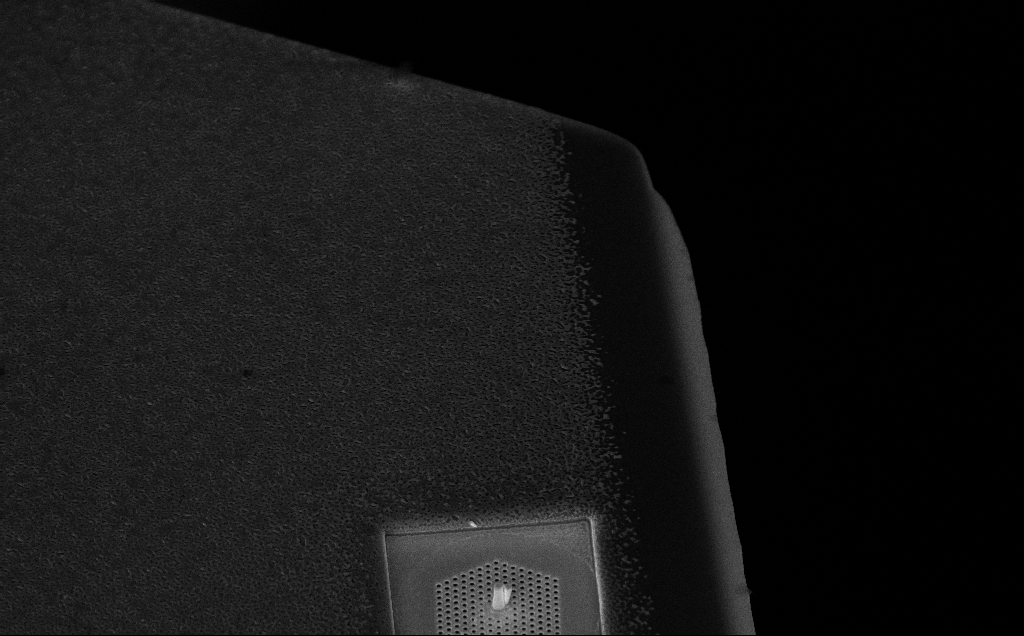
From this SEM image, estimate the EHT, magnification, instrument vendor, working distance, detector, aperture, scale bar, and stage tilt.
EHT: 10 kV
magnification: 12.28 K X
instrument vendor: Zeiss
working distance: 11 mm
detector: InLens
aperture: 30 µm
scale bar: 1000 nm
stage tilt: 45°